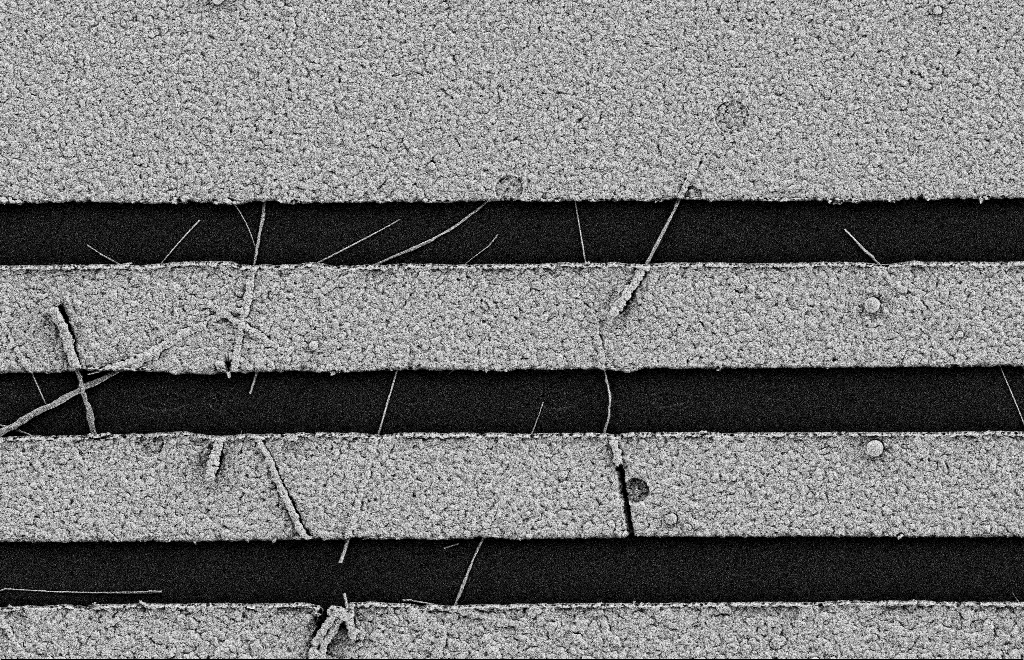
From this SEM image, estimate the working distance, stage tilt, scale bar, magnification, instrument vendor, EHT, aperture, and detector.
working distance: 11 mm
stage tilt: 0°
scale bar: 2000 nm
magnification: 15.45 K X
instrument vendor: Zeiss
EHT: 2 kV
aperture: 20 µm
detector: SE2